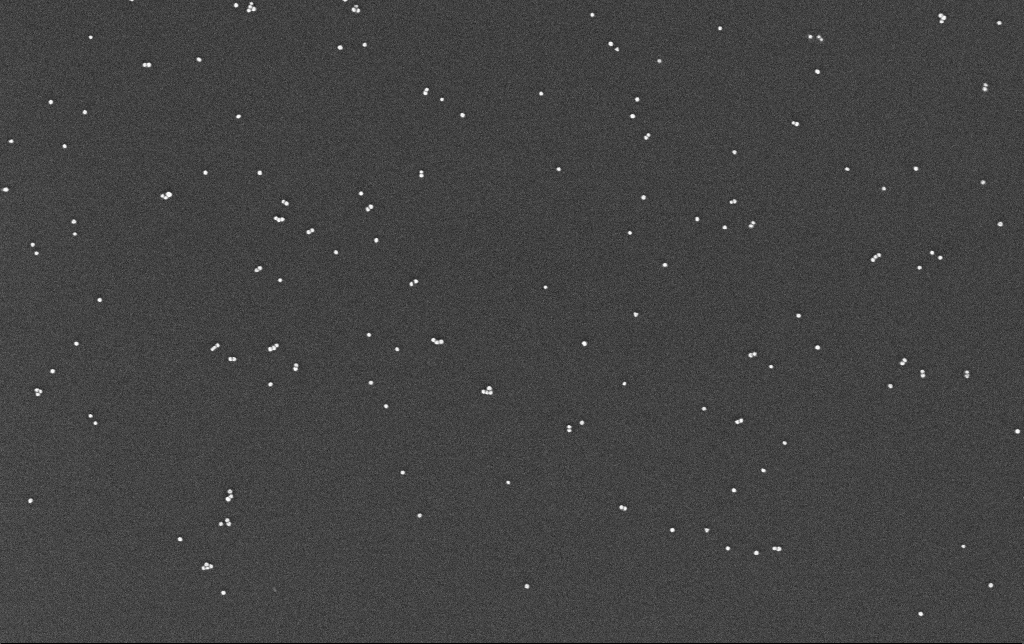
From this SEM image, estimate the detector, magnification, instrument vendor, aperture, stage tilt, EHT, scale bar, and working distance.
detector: InLens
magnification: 100 K X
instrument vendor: Zeiss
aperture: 30 µm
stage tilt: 0°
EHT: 10 kV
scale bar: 200 nm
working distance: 3.3 mm